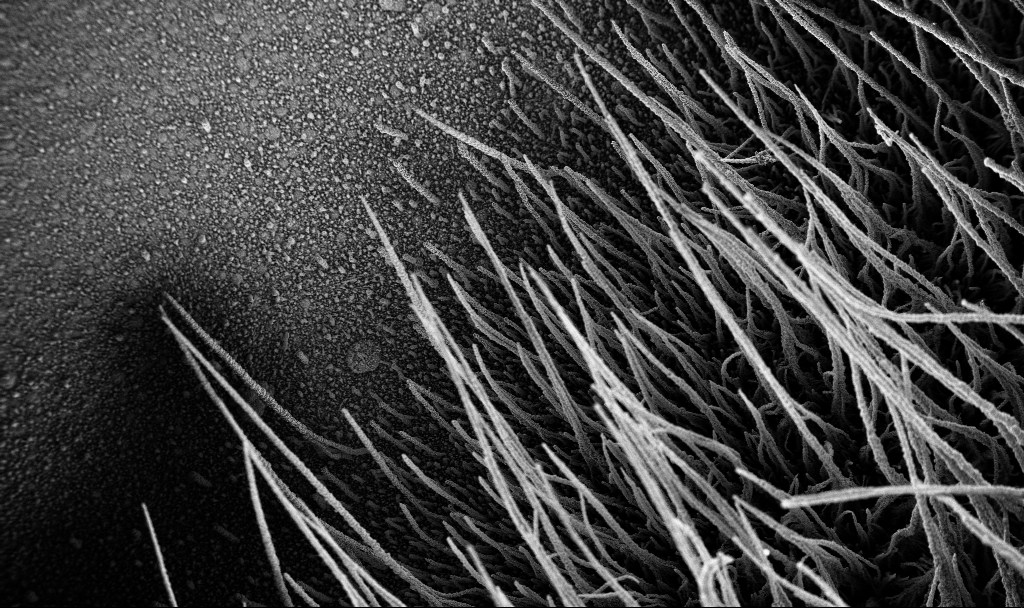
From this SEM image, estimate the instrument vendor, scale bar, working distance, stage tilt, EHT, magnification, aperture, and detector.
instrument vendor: Zeiss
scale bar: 100000 nm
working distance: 3.8 mm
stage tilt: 0°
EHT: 3 kV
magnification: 0.15 K X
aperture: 30 µm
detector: InLens